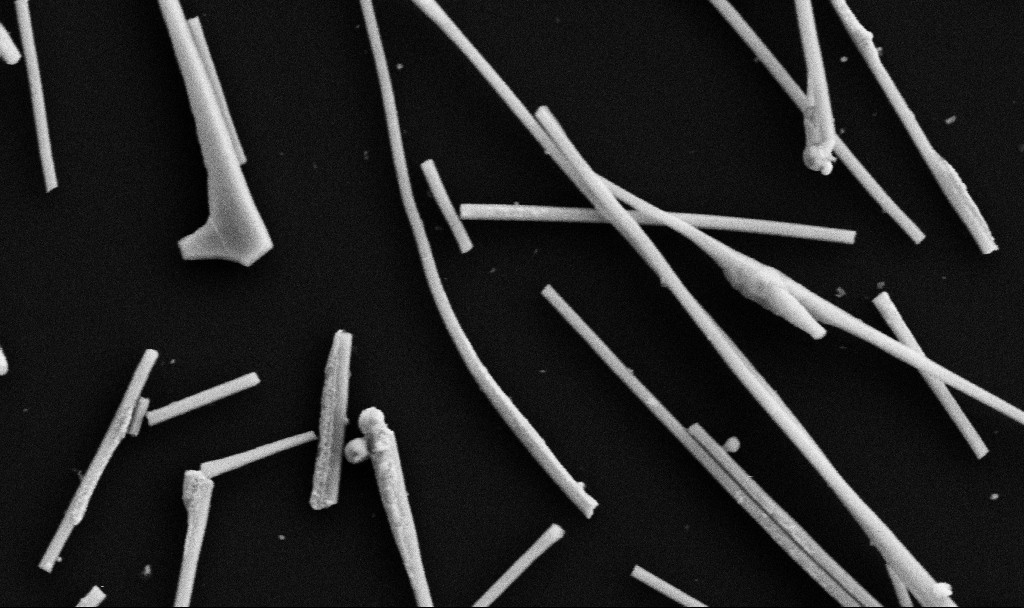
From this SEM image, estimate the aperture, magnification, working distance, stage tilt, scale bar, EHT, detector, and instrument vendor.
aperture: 30 µm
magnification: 50.39 K X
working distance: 10.7 mm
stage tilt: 0°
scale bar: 1000 nm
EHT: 5 kV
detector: SE2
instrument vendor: Zeiss